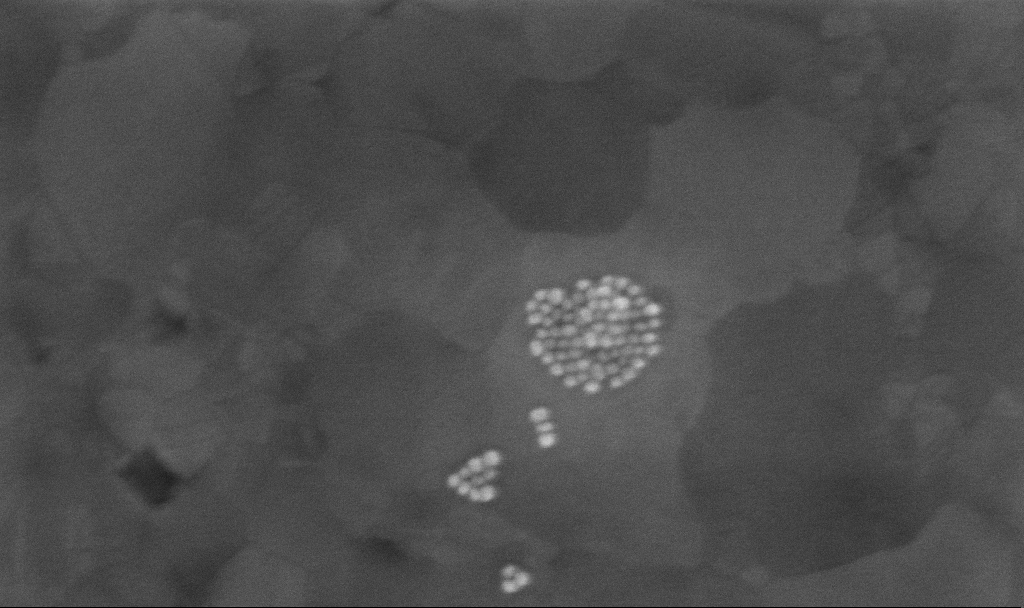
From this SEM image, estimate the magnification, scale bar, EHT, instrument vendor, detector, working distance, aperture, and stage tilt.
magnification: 300 K X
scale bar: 100 nm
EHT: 10 kV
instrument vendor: Zeiss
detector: InLens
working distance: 3.4 mm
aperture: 30 µm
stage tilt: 0°